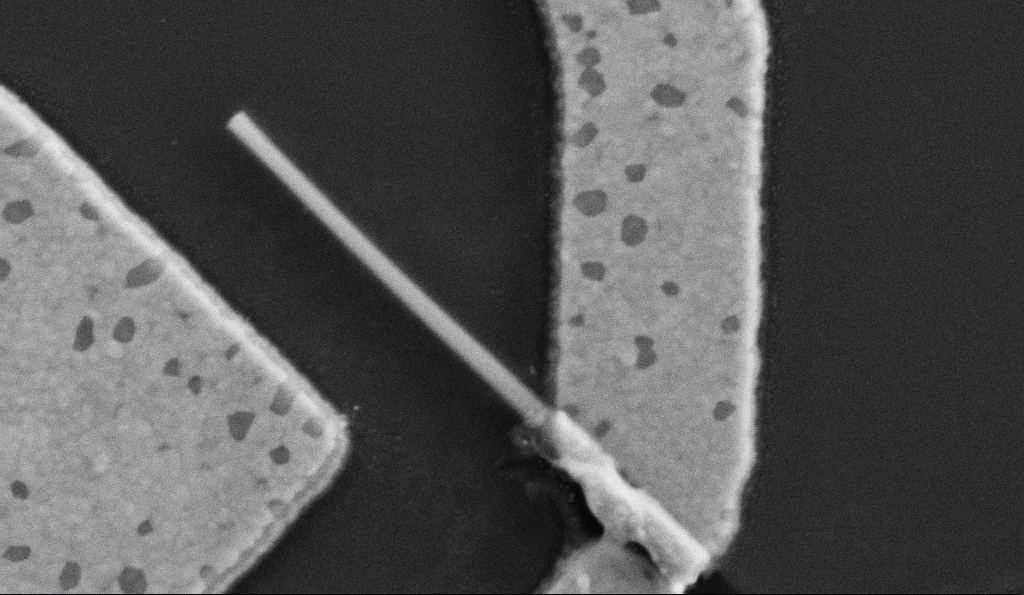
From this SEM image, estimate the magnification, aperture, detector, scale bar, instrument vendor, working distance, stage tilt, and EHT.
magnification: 100 K X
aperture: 30 µm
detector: SE2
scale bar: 200 nm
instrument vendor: Zeiss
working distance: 7.7 mm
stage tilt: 0°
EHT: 5 kV